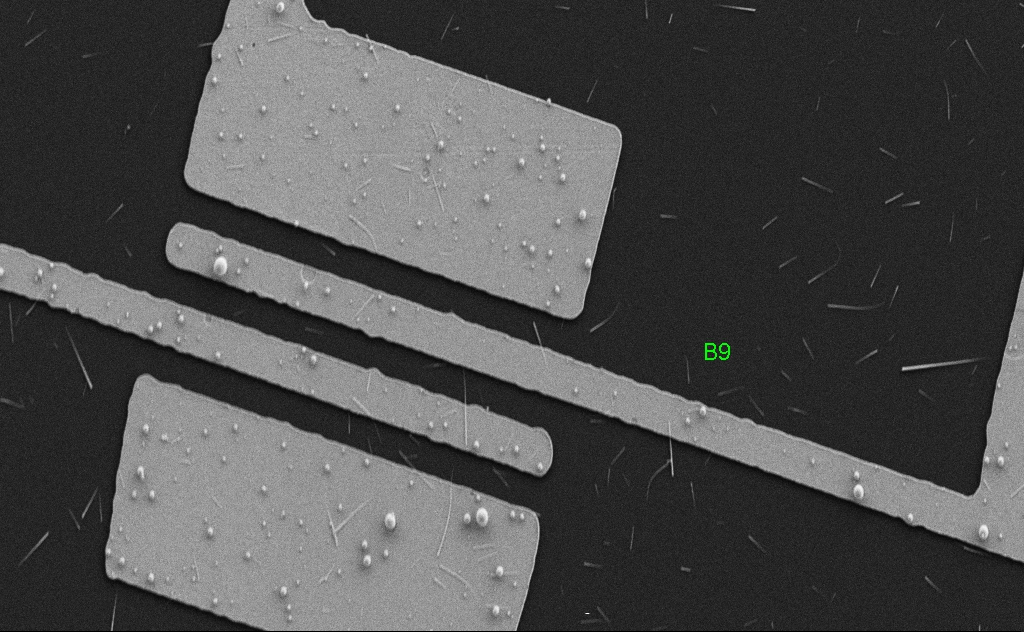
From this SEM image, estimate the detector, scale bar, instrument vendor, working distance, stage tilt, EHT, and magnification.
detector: SE2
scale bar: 10000 nm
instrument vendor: Zeiss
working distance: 5 mm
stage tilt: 0°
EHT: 5 kV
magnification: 5.5 K X